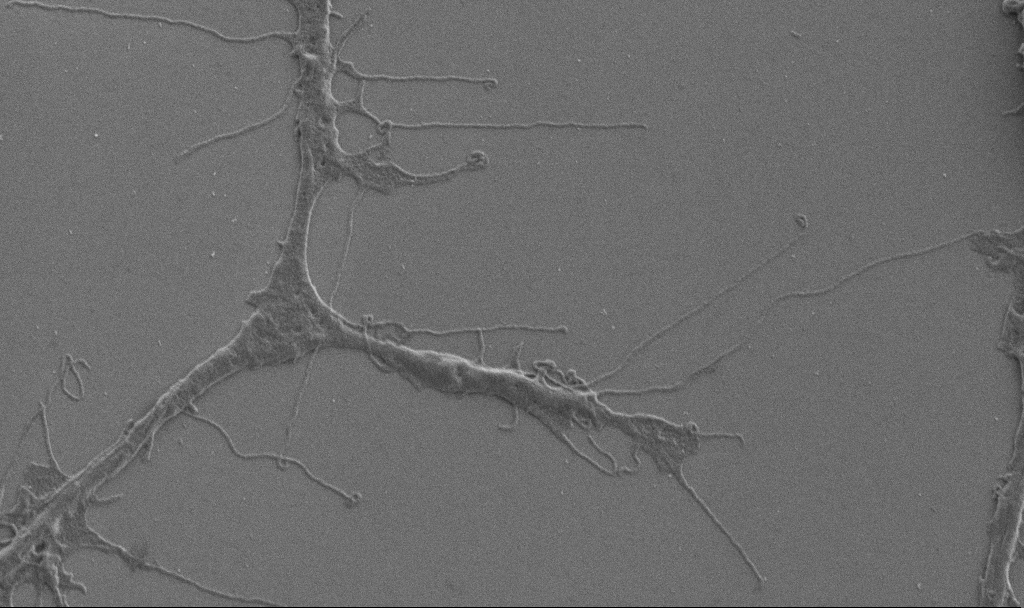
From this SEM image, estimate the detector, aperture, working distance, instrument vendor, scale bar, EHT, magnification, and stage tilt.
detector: SE2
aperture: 30 µm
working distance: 6.9 mm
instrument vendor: Zeiss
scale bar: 2000 nm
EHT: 1 kV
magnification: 10 K X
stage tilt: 0°